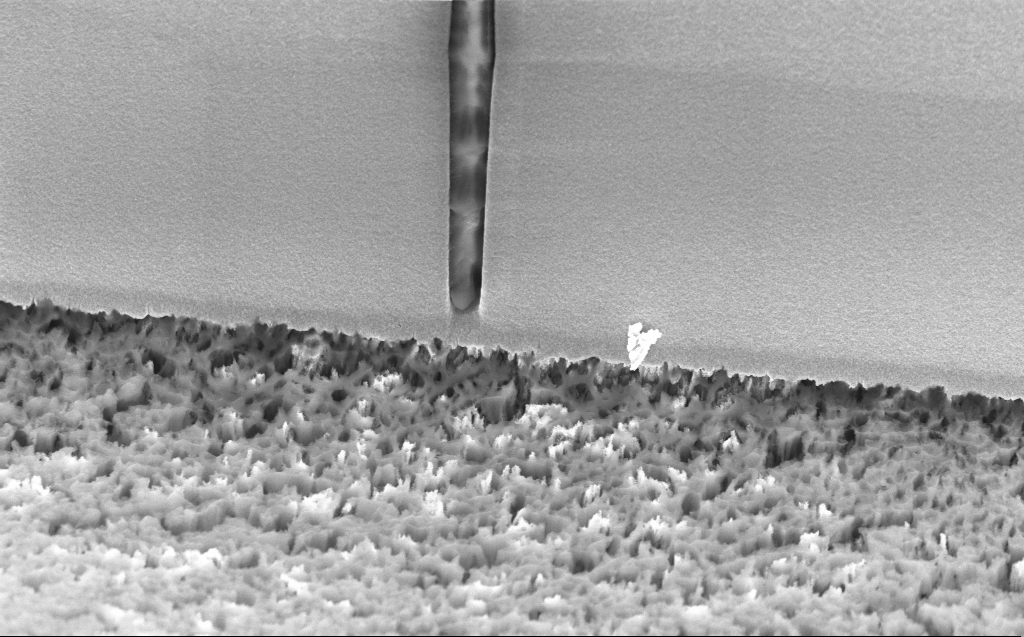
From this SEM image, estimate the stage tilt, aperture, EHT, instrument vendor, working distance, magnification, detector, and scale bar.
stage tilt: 45°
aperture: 30 µm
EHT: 5 kV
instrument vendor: Zeiss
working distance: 5 mm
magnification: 11.46 K X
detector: InLens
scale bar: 2000 nm